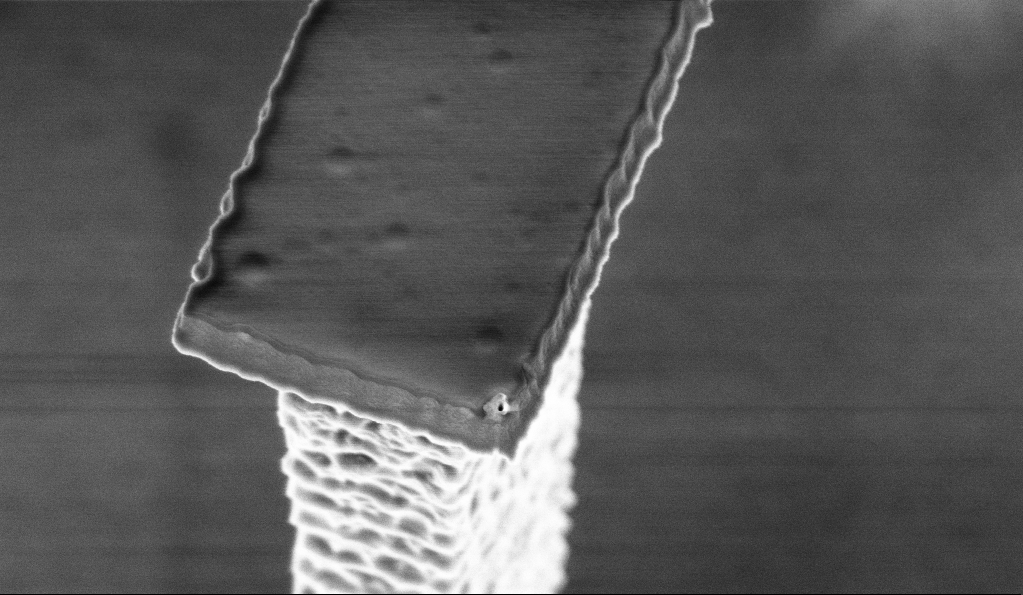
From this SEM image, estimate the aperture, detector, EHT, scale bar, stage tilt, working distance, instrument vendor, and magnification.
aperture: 30 µm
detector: InLens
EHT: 3 kV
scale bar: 1000 nm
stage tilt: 30°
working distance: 5.7 mm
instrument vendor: Zeiss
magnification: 48.12 K X